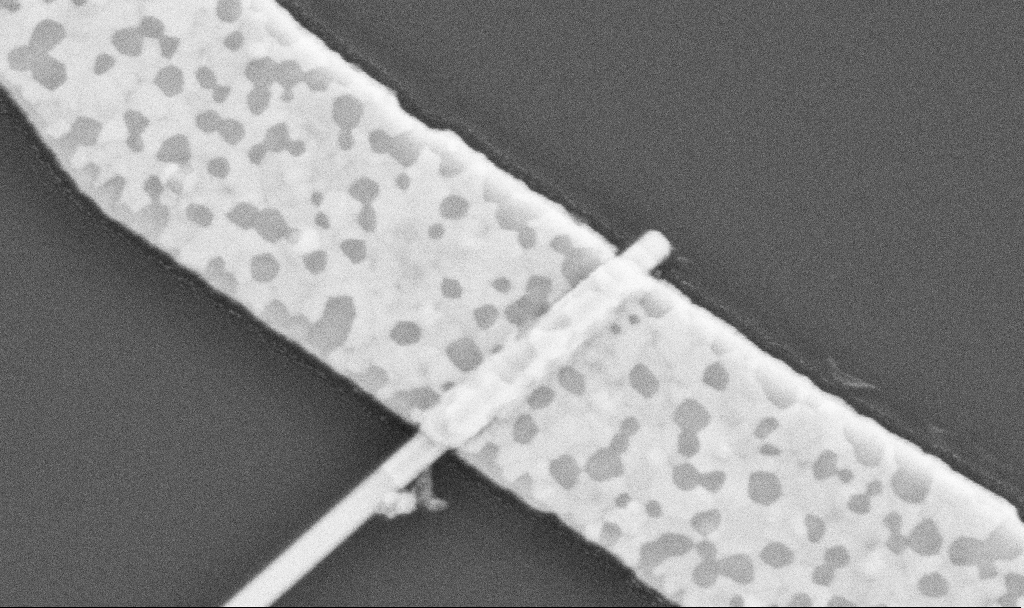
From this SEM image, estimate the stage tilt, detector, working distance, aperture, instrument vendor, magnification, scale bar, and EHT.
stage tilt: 0°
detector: SE2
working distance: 10.5 mm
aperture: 30 µm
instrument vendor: Zeiss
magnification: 100 K X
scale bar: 200 nm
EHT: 5 kV